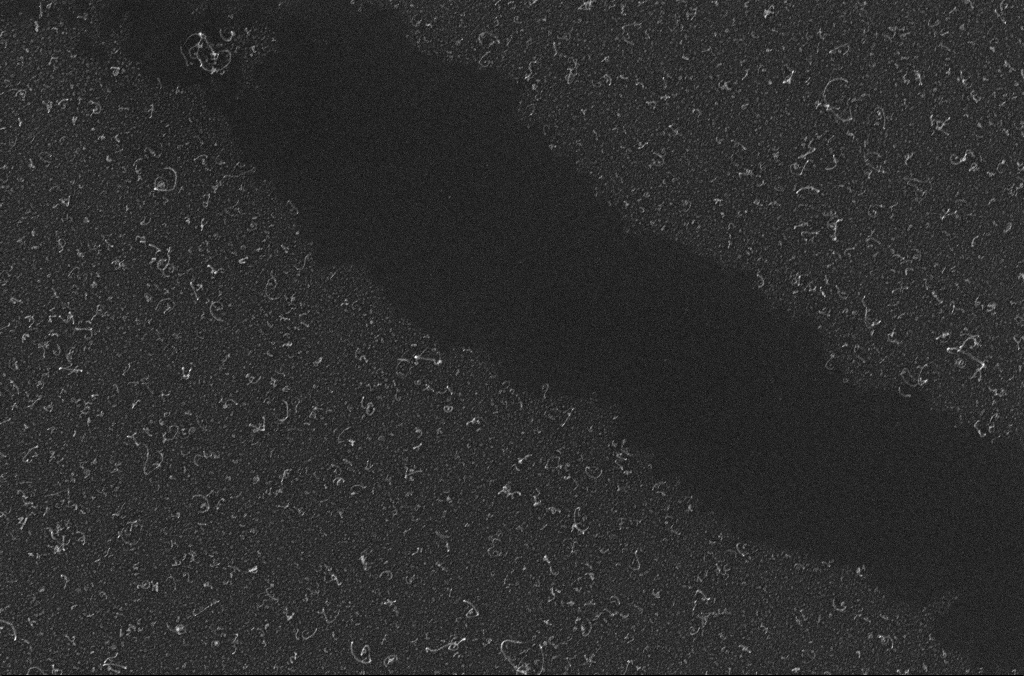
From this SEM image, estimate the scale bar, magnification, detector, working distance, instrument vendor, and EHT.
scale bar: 1000 nm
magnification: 50 K X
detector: InLens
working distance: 3.3 mm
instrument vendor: Zeiss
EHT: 10 kV